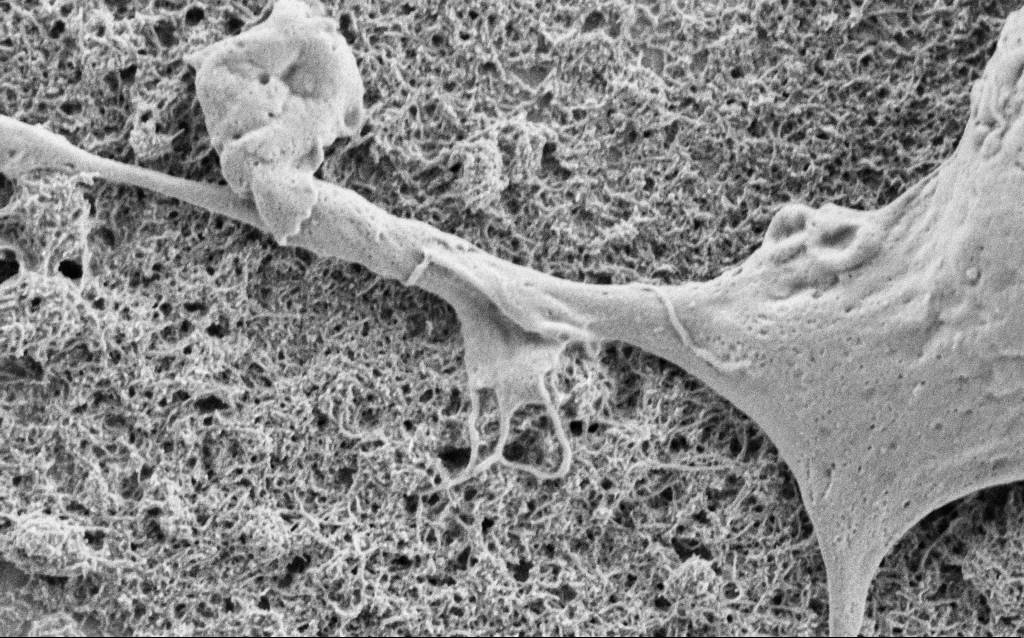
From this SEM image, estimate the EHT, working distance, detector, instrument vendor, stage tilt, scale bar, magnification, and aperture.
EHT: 1.5 kV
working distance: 6.7 mm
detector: SE2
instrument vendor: Zeiss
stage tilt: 0°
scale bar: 1000 nm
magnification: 25 K X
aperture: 30 µm